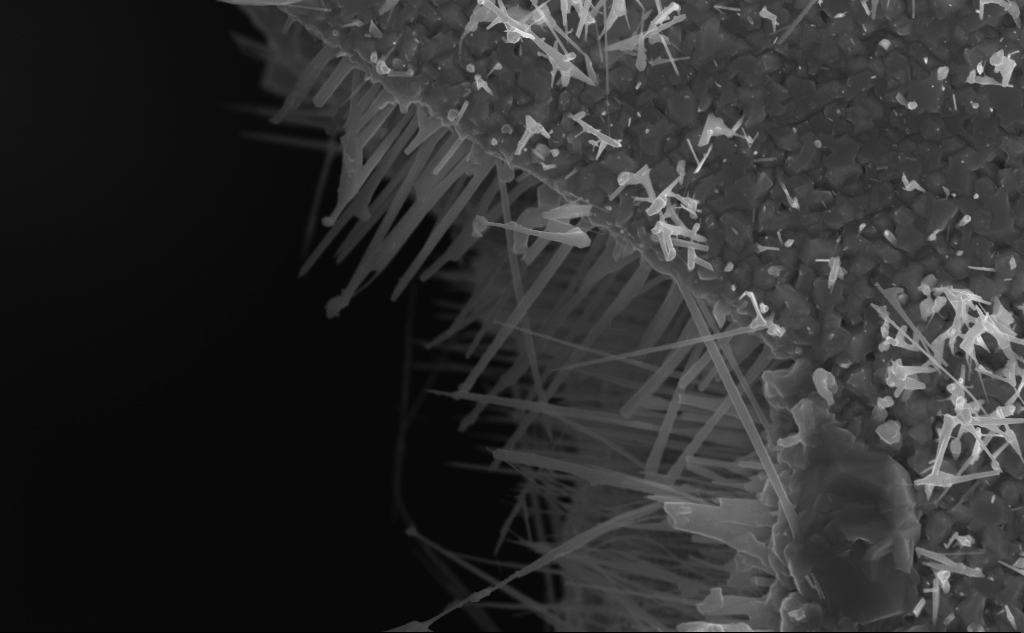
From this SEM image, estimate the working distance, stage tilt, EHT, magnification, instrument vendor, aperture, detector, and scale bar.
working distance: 6 mm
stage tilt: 0°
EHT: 10 kV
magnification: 26.85 K X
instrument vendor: Zeiss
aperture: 30 µm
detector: InLens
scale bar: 1000 nm